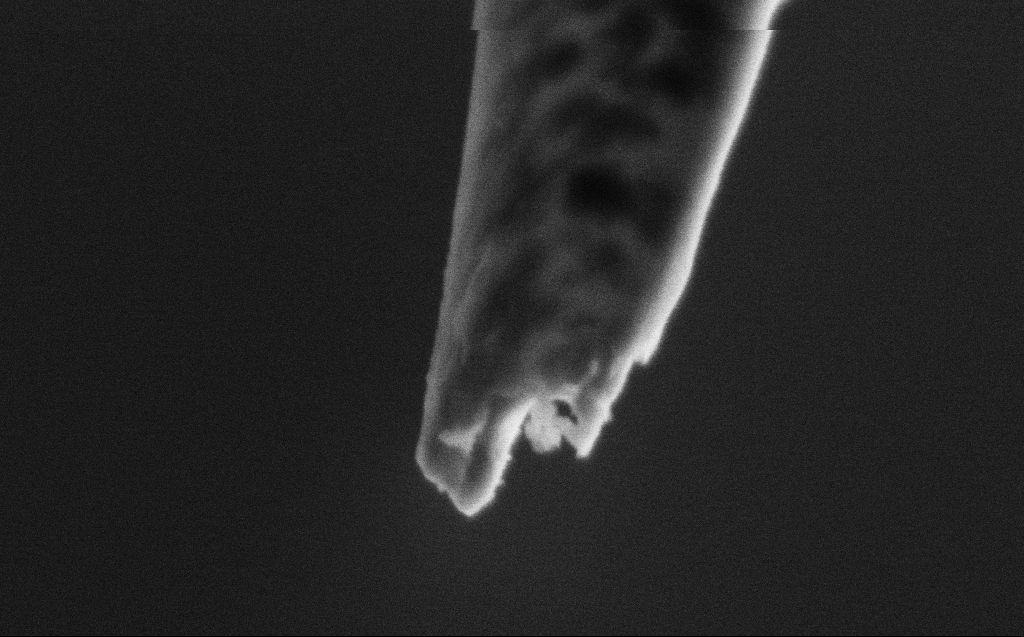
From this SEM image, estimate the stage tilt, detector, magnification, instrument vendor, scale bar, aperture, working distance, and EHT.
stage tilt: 45°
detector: SE2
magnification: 250 K X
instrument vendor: Zeiss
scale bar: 200 nm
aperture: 30 µm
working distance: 4 mm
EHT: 2 kV